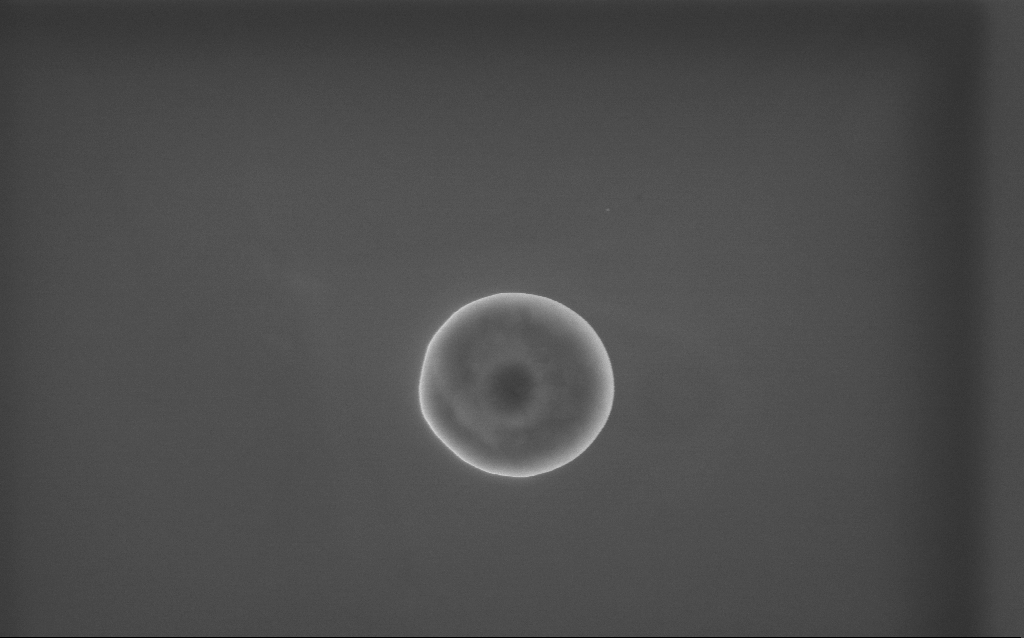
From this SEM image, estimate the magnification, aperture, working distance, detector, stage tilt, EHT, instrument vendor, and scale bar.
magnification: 57 K X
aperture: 30 µm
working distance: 2 mm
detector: InLens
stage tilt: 0°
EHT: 10 kV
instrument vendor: Zeiss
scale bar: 1000 nm